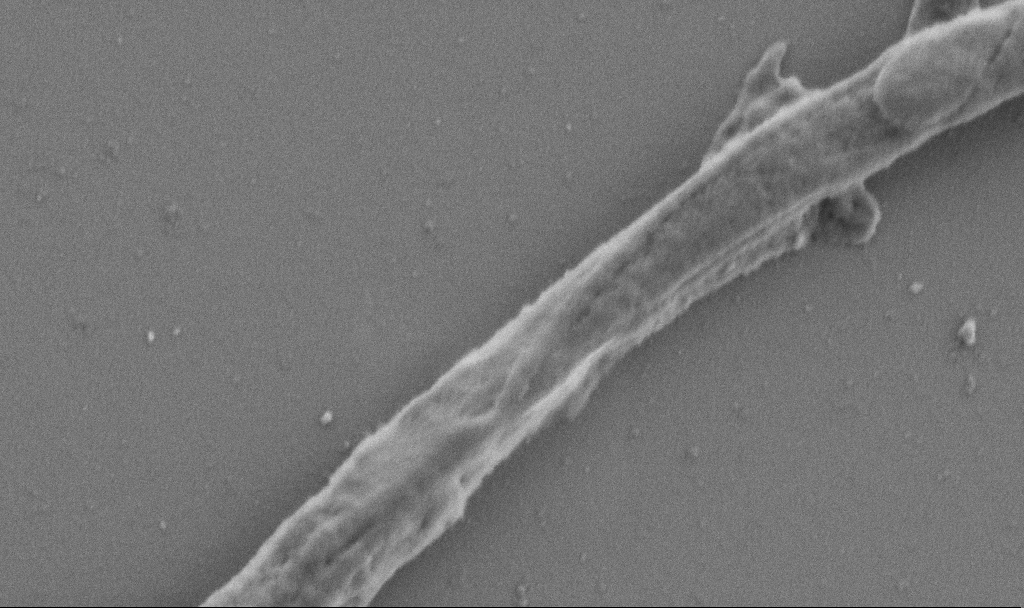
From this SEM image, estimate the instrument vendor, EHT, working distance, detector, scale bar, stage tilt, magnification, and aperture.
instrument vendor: Zeiss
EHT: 1 kV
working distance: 6.9 mm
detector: SE2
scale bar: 1000 nm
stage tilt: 0°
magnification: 50 K X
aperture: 30 µm